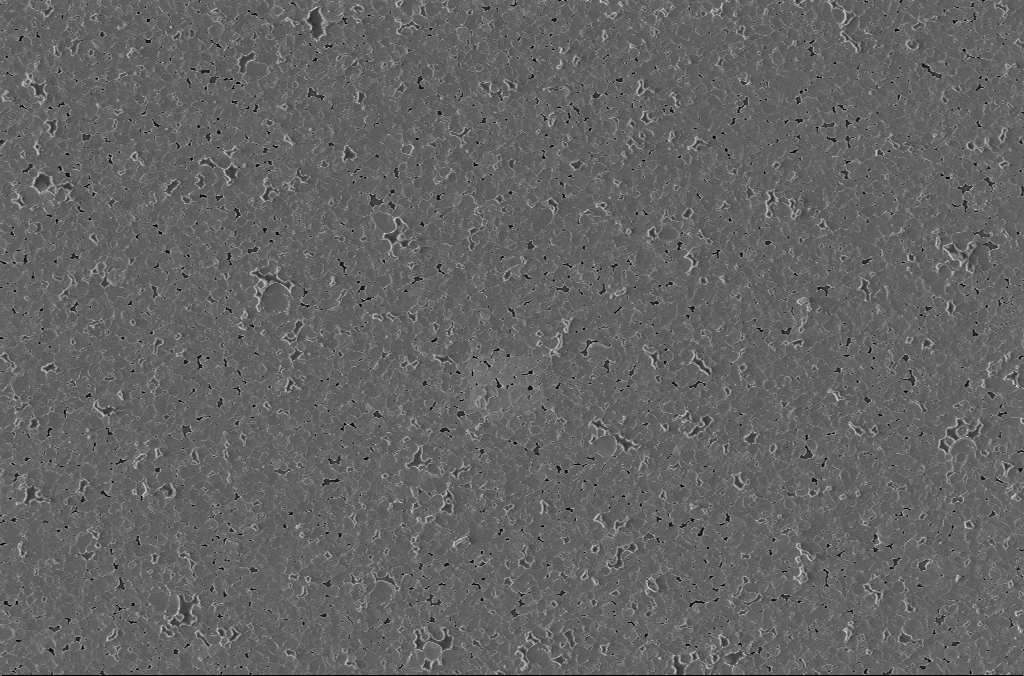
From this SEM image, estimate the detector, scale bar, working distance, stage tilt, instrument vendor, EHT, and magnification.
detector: InLens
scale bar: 10000 nm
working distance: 3 mm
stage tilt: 0°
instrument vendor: Zeiss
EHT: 2 kV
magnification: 5 K X